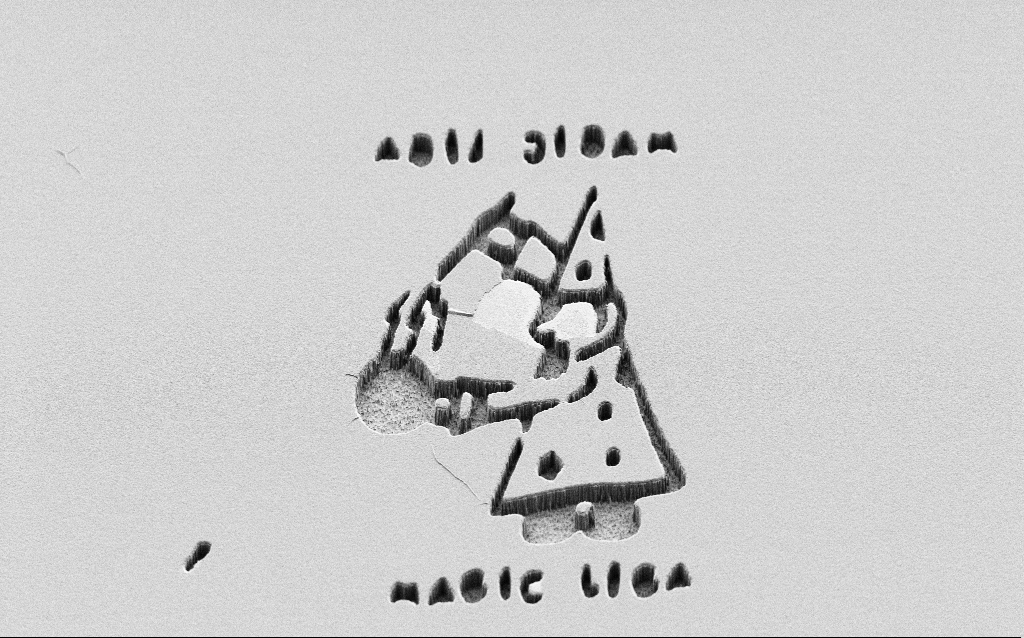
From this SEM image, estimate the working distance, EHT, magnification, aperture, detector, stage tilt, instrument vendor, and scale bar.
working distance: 8 mm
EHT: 2 kV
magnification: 1.24 K X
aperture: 30 µm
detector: SE2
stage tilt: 45°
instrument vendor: Zeiss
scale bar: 20000 nm